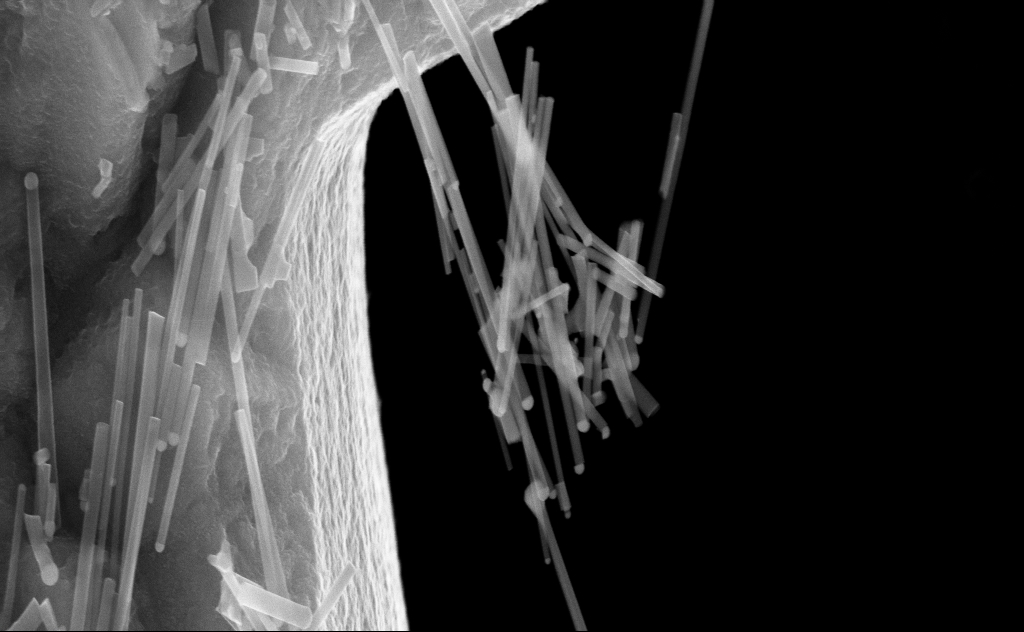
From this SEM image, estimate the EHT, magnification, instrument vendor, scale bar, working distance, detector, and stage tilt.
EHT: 20 kV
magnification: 66.11 K X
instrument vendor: Zeiss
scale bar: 1000 nm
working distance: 8 mm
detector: InLens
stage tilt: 0°